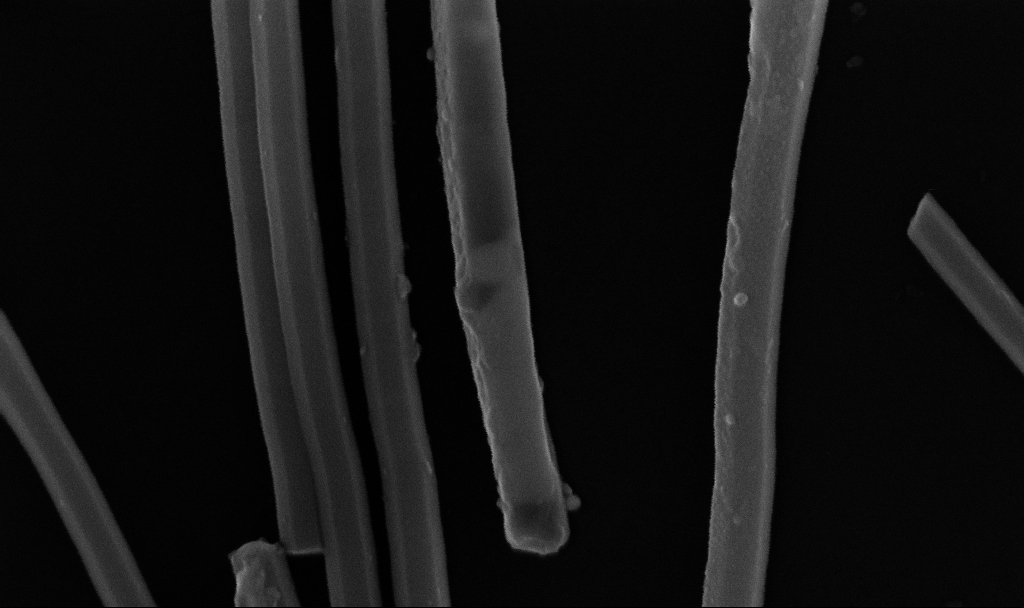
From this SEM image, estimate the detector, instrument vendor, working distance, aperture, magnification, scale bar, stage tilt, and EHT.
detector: InLens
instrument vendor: Zeiss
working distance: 6.7 mm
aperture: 30 µm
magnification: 185.32 K X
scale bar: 200 nm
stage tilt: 0°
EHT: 10 kV